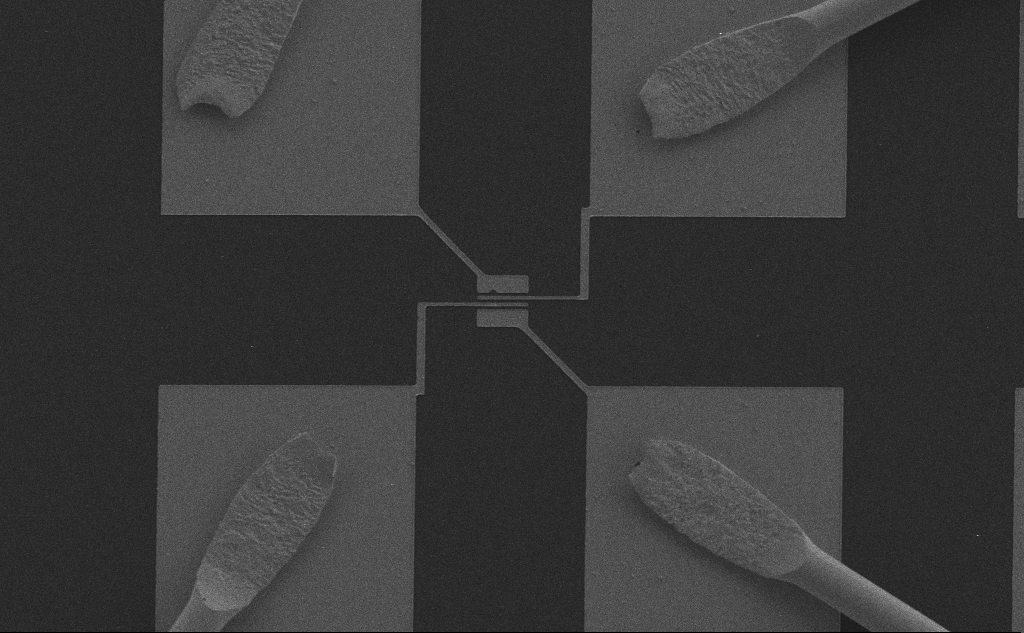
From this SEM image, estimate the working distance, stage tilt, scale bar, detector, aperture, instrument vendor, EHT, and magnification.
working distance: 6 mm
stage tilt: -0.1°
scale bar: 100000 nm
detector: SE2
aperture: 30 µm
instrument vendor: Zeiss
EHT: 10 kV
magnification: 0.628 K X